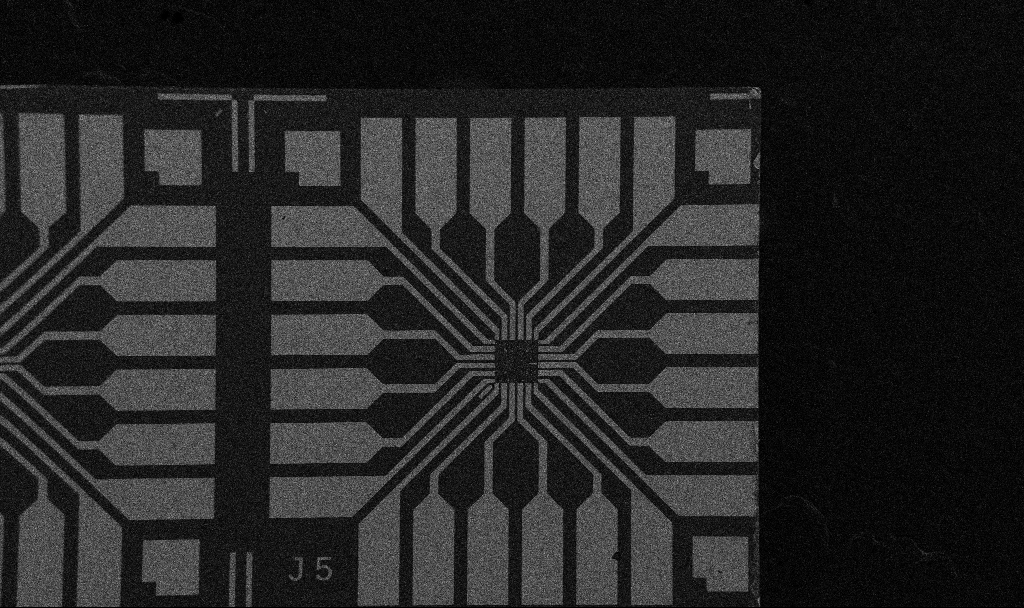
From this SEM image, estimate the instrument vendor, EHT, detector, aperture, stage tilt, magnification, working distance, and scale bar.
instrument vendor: Zeiss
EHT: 5 kV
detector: SE2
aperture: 30 µm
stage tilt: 0°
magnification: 0.1 K X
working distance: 10.7 mm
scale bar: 200000 nm